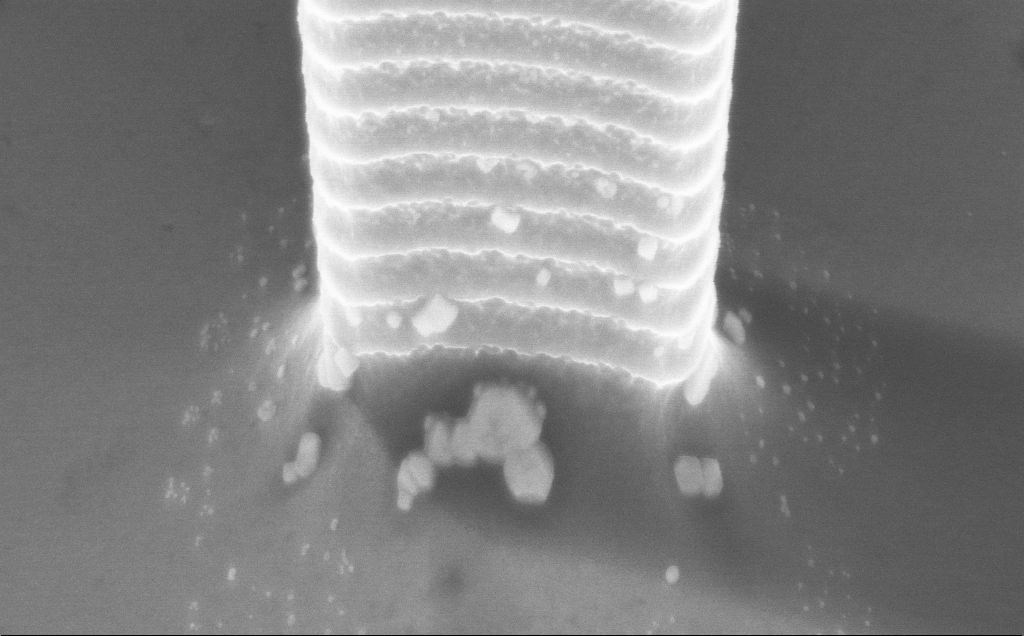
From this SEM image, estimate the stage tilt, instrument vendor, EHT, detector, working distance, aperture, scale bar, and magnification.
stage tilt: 45°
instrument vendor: Zeiss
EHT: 7.5 kV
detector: InLens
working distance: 5 mm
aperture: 30 µm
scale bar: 1000 nm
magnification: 45.15 K X